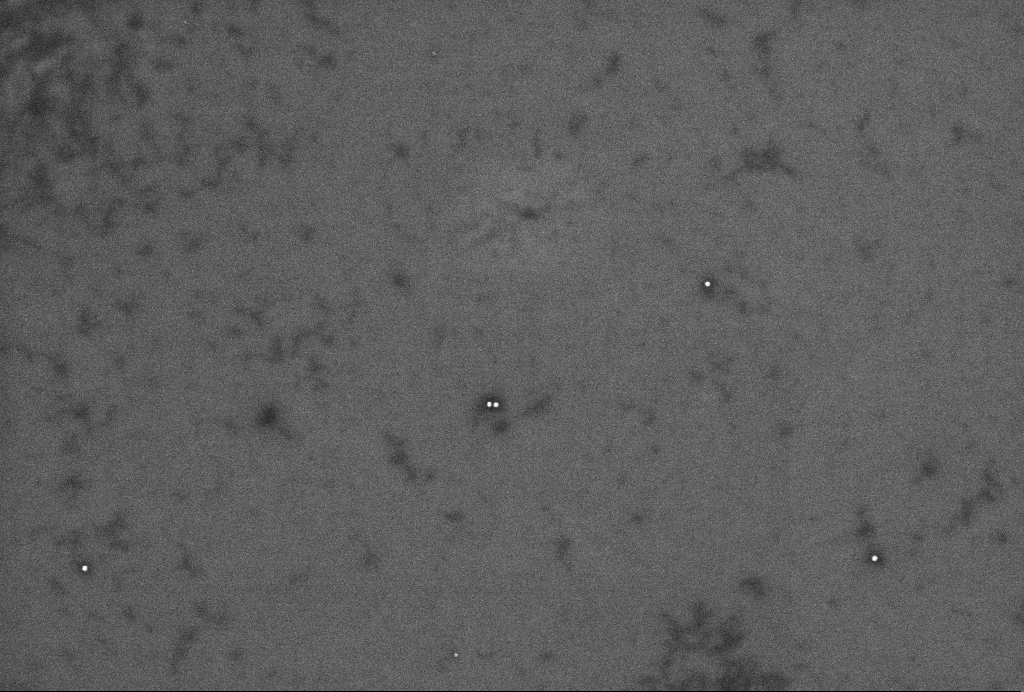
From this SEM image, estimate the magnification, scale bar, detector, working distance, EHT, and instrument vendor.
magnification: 64.42 K X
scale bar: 200 nm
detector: InLens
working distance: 3.3 mm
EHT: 2 kV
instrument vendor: Zeiss